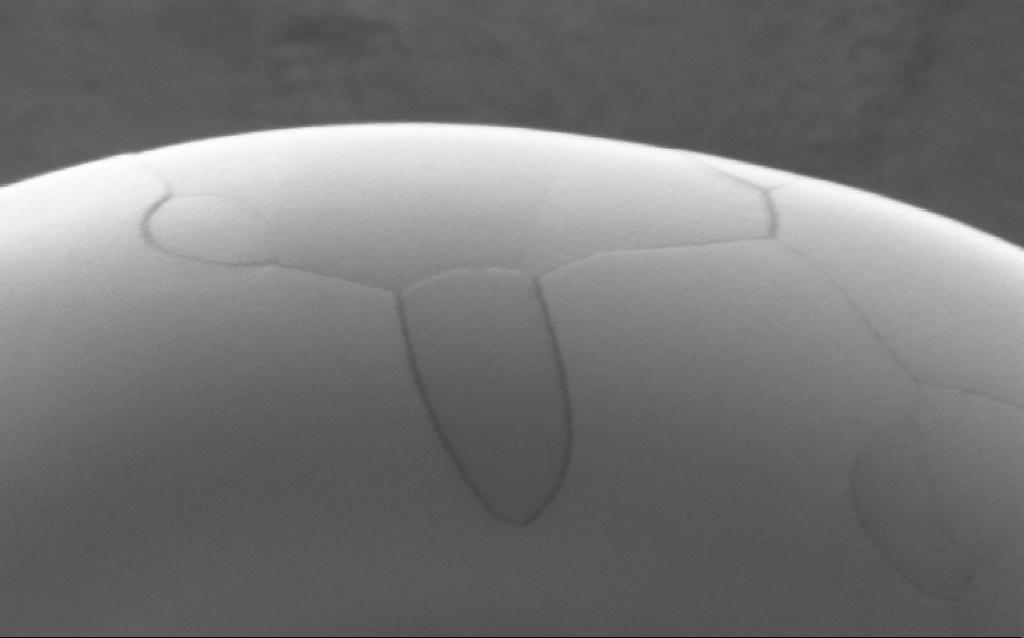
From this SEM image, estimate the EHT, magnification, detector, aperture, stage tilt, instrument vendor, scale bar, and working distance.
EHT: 5 kV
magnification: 236.7 K X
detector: InLens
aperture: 30 µm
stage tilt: -0°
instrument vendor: Zeiss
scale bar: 200 nm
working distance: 2 mm